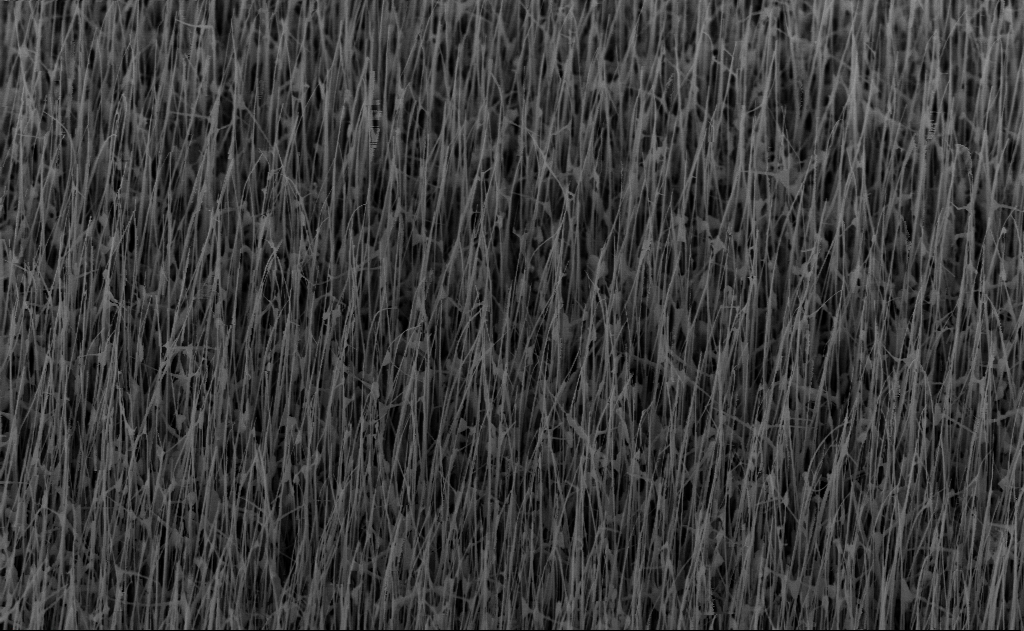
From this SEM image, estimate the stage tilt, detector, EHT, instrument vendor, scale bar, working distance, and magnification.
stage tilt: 45°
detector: InLens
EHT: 10 kV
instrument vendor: Zeiss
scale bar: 2000 nm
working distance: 7 mm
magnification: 20 K X